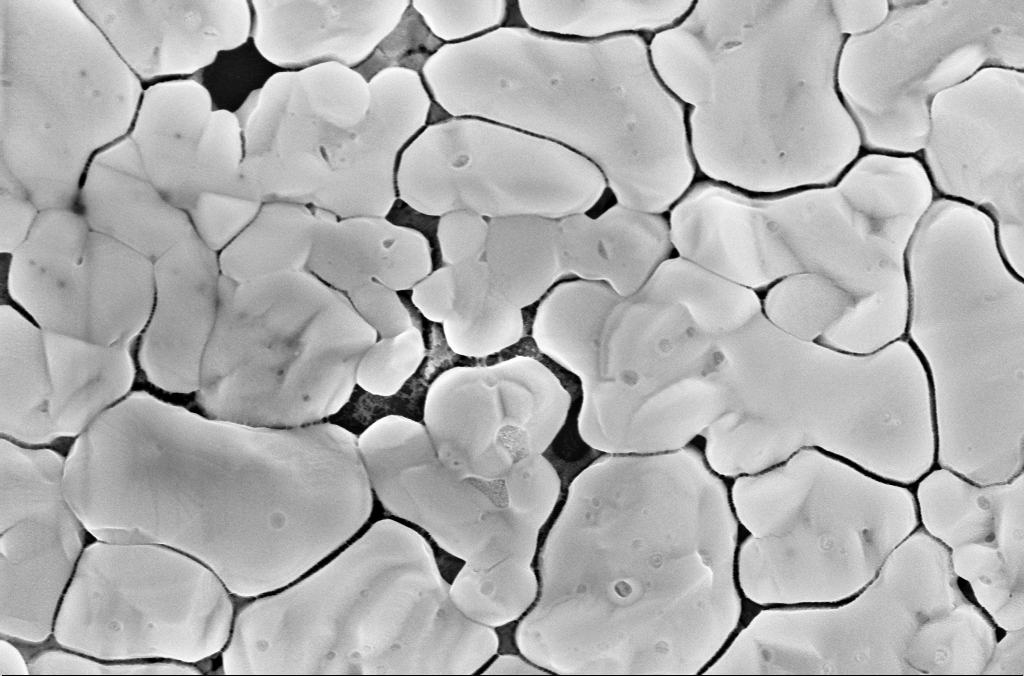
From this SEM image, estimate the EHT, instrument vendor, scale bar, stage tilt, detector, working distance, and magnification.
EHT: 5 kV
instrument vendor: Zeiss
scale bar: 200 nm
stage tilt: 0°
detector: InLens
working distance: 3.1 mm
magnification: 70 K X